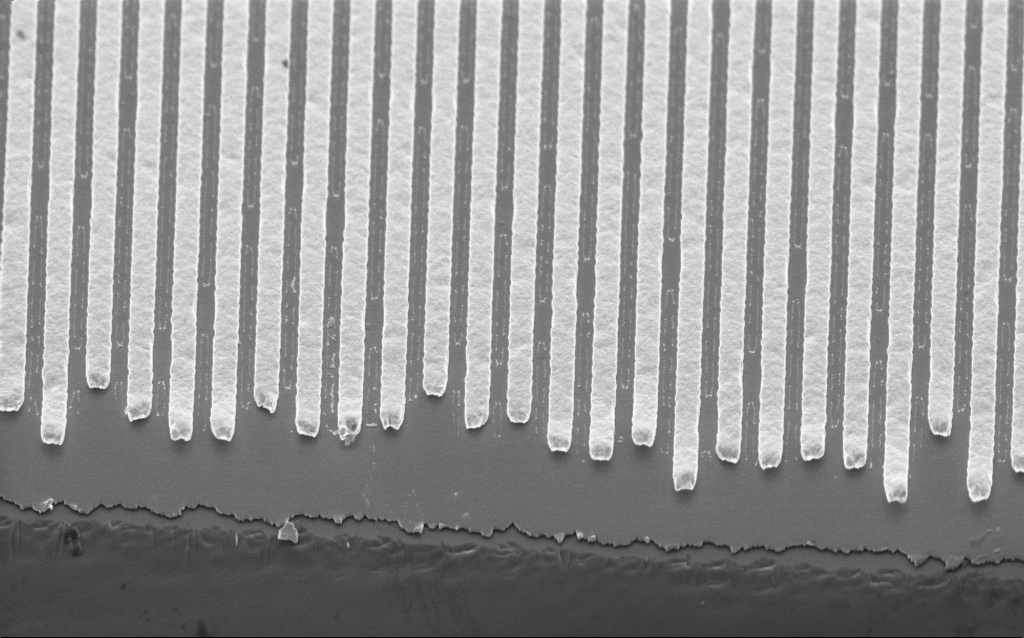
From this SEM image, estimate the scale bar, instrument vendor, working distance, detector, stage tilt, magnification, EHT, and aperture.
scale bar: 2000 nm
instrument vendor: Zeiss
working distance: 3.4 mm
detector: InLens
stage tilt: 45°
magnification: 31.15 K X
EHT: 2 kV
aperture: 30 µm